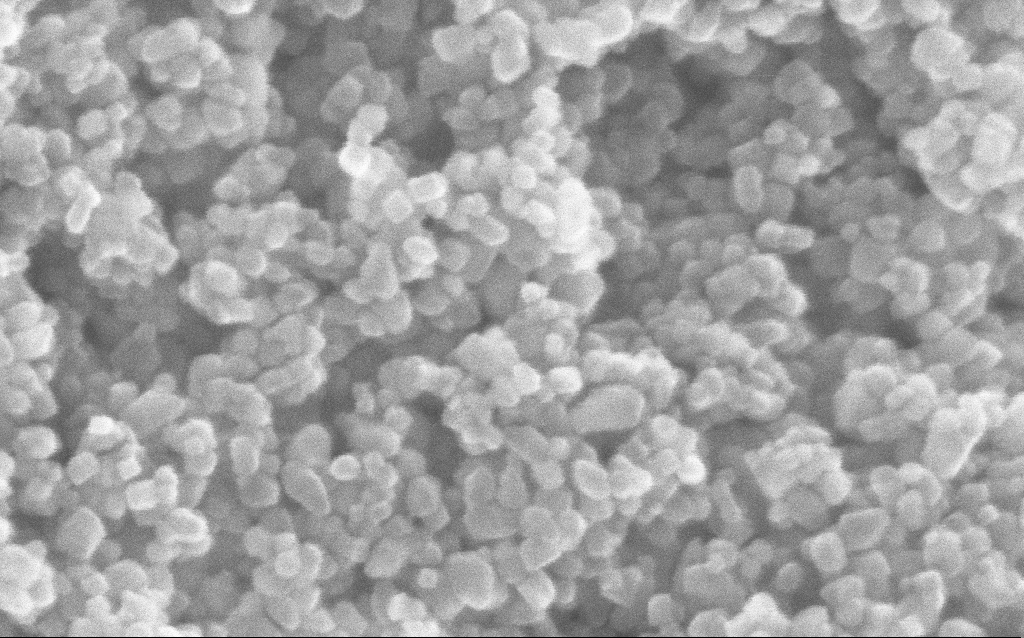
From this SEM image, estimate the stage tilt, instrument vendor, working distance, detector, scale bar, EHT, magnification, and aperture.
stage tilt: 0°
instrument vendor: Zeiss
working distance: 3.8 mm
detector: InLens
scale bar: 100 nm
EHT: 10 kV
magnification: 477.61 K X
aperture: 30 µm